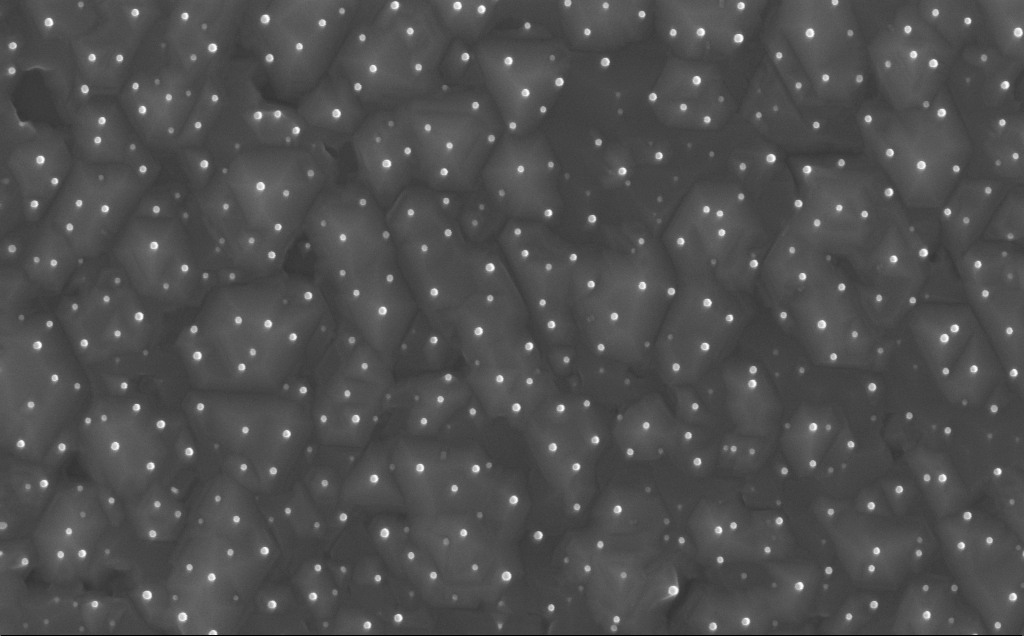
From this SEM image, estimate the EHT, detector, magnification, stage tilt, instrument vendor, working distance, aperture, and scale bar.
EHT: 10 kV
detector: InLens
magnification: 80 K X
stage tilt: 0°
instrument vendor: Zeiss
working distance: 6 mm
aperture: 30 µm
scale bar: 200 nm